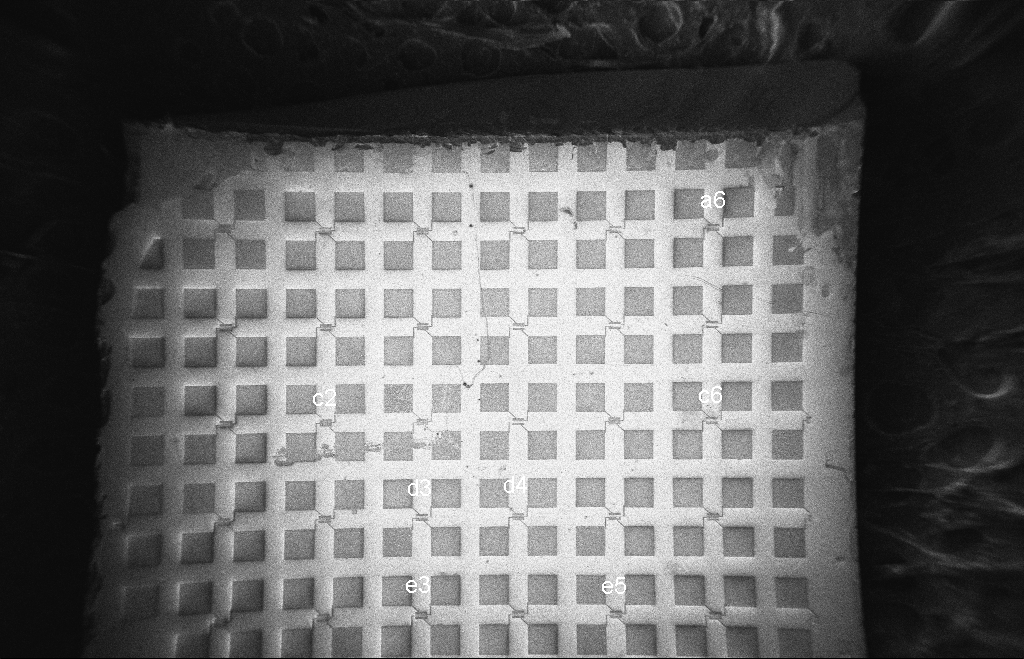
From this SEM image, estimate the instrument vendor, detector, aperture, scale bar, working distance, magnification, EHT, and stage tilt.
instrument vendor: Zeiss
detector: InLens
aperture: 20 µm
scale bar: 200000 nm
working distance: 8 mm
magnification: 0.071 K X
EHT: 5 kV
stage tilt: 0°